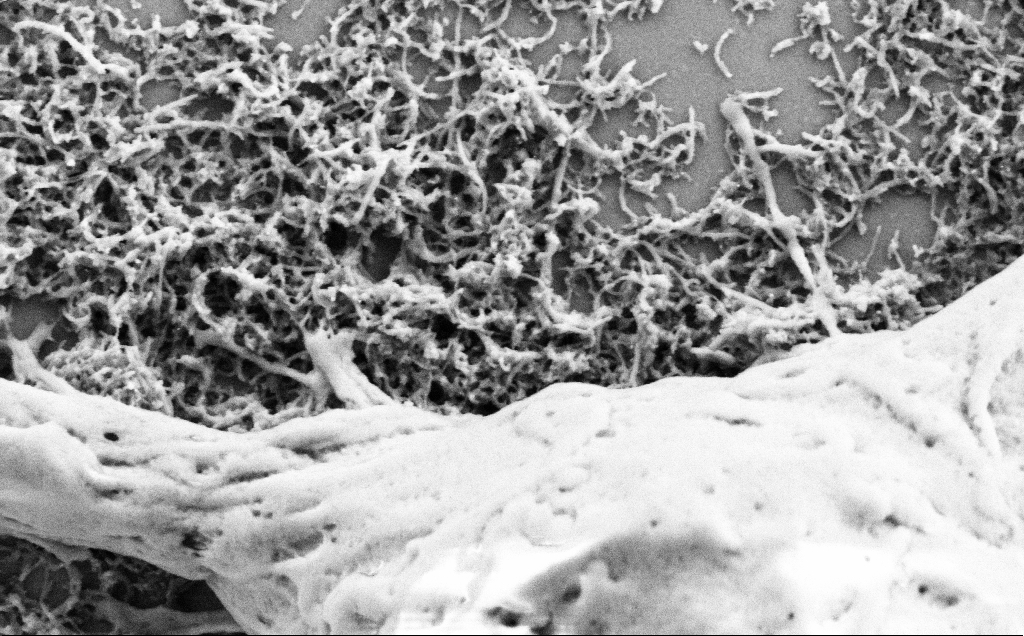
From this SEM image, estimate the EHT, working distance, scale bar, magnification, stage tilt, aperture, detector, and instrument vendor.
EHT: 2 kV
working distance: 7.1 mm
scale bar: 1000 nm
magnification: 50 K X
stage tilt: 0°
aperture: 30 µm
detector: SE2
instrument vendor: Zeiss